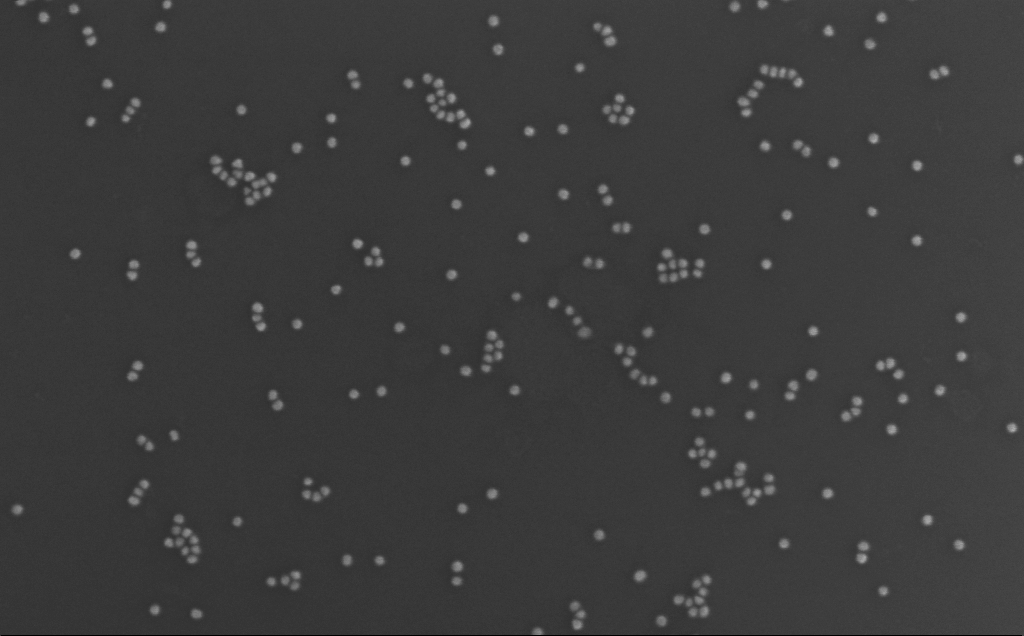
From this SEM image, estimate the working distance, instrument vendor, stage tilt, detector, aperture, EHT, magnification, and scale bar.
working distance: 3 mm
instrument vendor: Zeiss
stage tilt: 0°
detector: InLens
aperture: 30 µm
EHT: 10 kV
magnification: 210.33 K X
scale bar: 200 nm